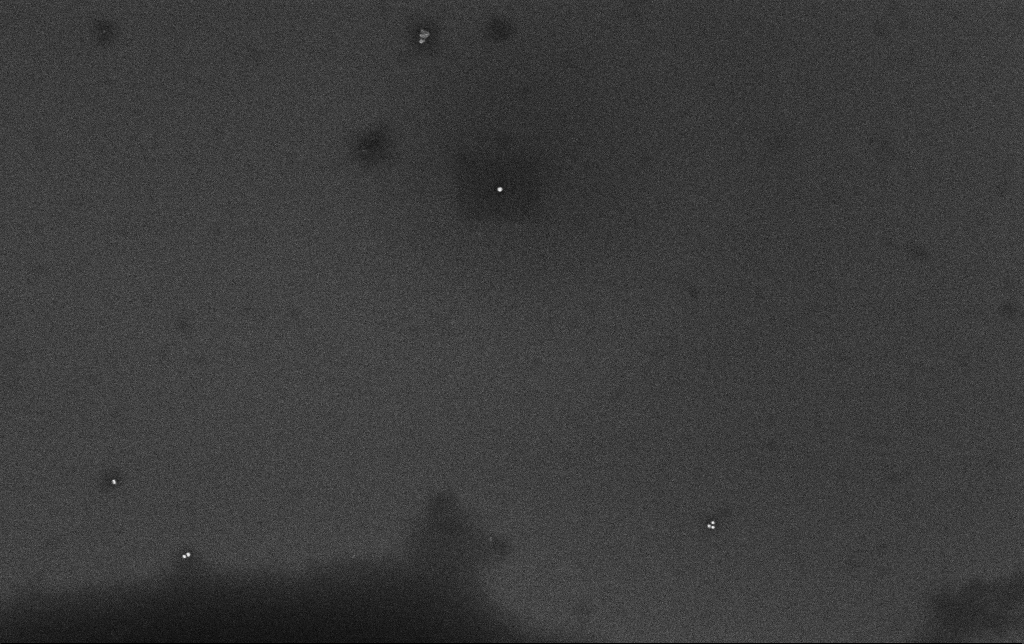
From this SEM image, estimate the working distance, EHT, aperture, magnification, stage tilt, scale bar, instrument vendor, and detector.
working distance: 3.4 mm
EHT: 10 kV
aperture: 30 µm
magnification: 100 K X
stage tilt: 0°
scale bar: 200 nm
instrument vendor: Zeiss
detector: InLens